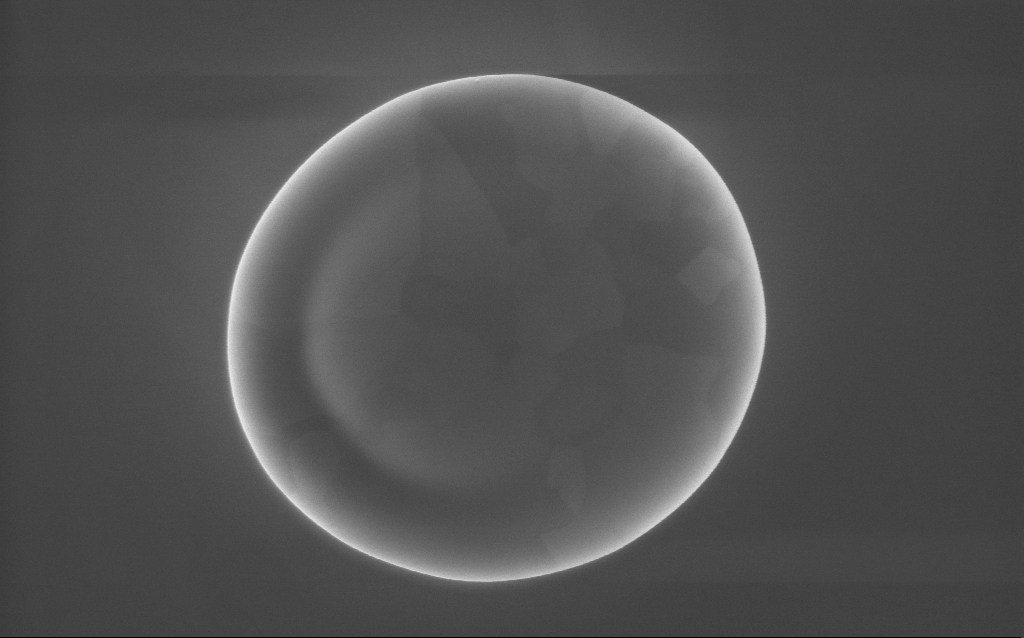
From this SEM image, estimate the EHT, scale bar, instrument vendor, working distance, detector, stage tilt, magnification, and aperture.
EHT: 10 kV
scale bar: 1000 nm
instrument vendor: Zeiss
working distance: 3 mm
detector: InLens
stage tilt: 0°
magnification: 58 K X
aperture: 30 µm